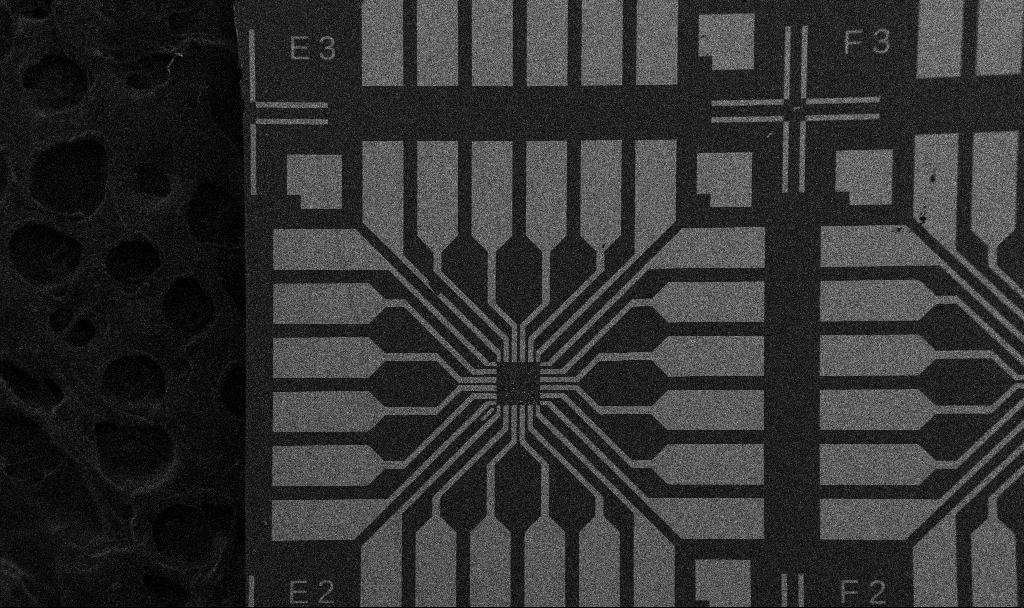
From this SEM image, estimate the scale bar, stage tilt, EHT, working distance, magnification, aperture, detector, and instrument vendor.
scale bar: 200000 nm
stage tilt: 0°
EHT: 5 kV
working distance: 10.7 mm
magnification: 0.1 K X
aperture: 30 µm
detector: SE2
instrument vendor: Zeiss